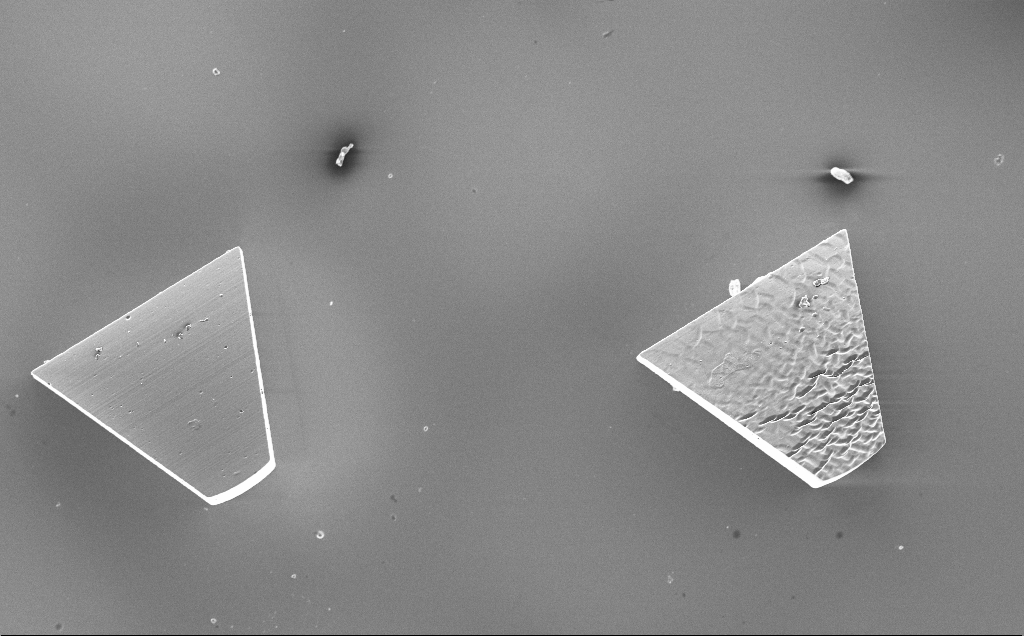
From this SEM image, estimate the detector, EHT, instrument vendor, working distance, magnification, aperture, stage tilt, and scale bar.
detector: InLens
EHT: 10 kV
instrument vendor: Zeiss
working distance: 10 mm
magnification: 0.227 K X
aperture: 30 µm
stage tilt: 0°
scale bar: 100000 nm